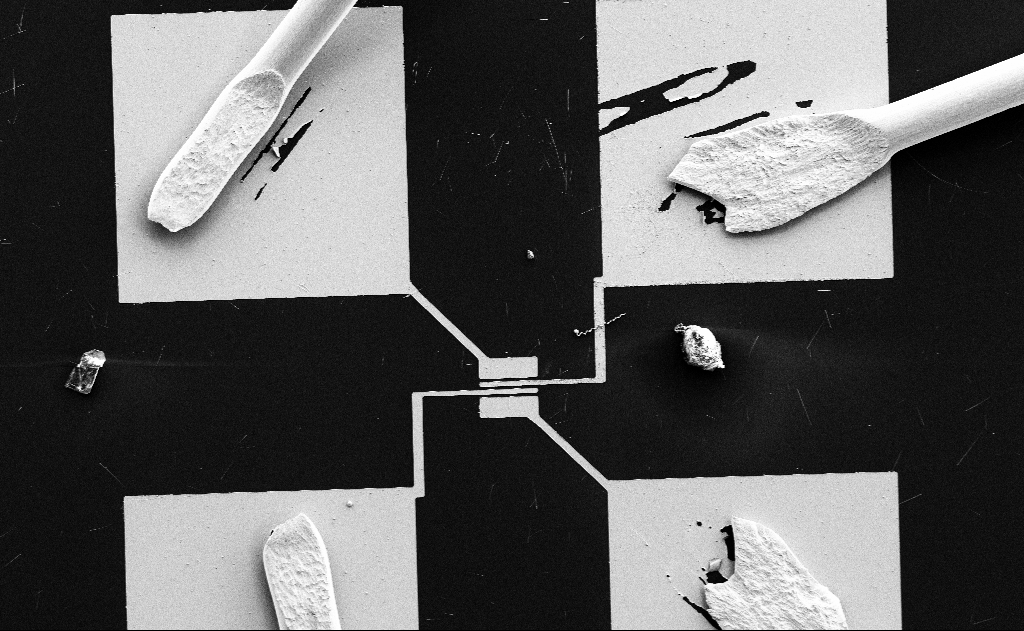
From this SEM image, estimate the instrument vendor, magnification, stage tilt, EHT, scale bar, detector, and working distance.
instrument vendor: Zeiss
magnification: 0.712 K X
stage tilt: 0°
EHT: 5 kV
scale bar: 100000 nm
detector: SE2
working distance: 15 mm